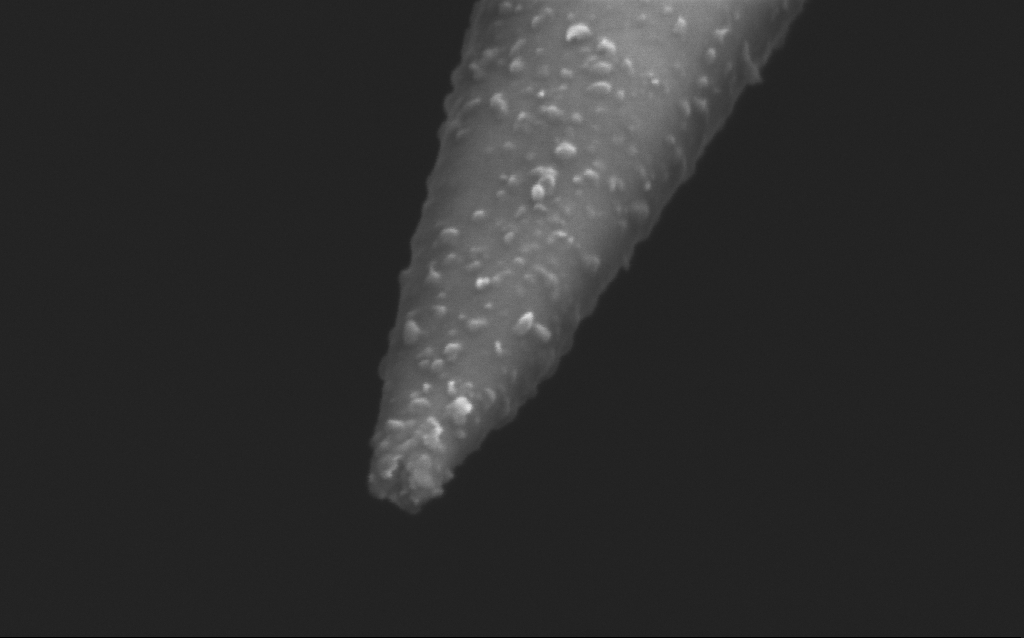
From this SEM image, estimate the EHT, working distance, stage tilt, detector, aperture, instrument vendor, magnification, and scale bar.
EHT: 2.5 kV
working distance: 5 mm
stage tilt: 45°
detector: InLens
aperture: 30 µm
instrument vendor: Zeiss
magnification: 250 K X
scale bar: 200 nm